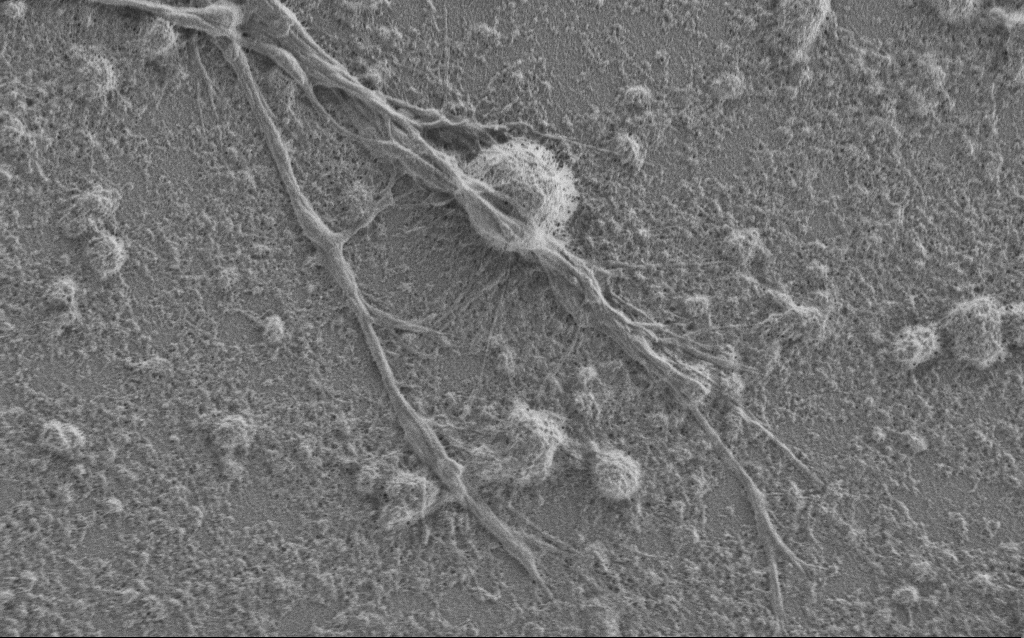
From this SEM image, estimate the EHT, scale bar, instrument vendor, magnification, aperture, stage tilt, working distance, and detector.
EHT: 1 kV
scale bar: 2000 nm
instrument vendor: Zeiss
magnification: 7.5 K X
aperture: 30 µm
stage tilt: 0°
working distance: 6 mm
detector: SE2